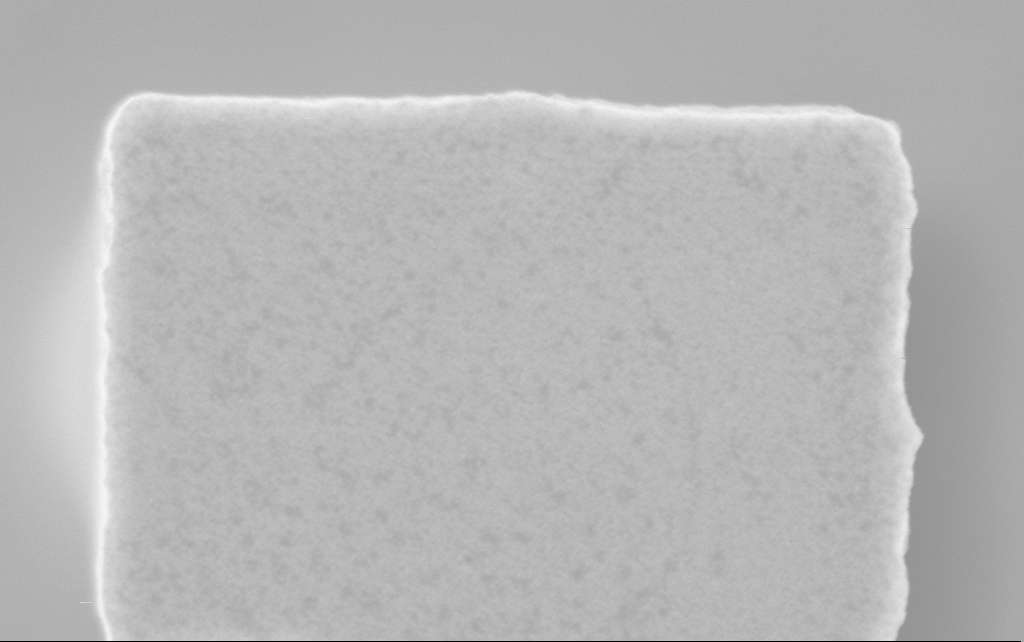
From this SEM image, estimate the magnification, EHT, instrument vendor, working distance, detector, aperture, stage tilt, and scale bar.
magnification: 96.94 K X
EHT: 3 kV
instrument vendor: Zeiss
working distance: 2.5 mm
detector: InLens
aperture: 30 µm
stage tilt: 0°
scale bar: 200 nm